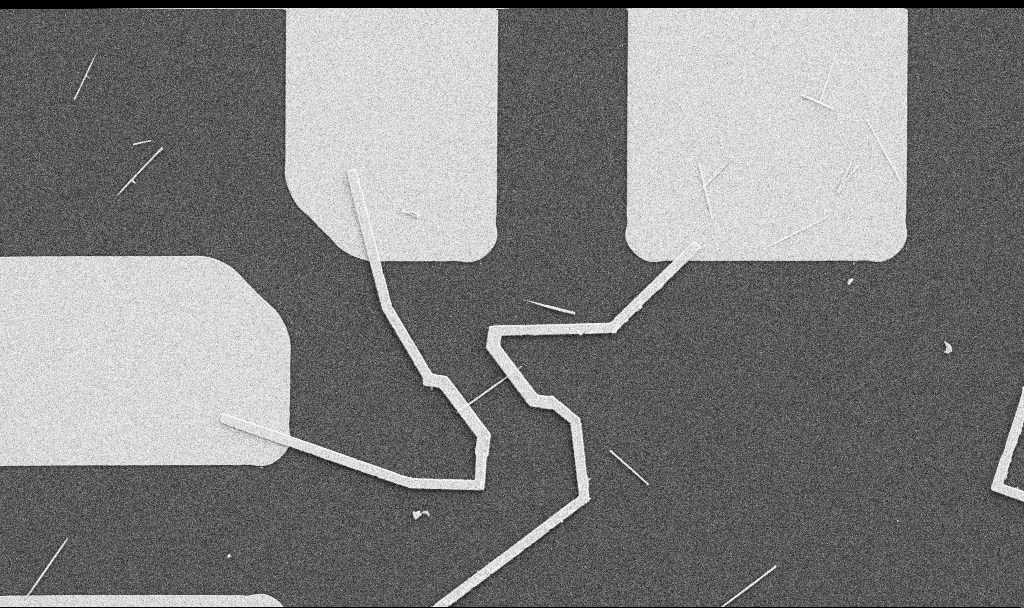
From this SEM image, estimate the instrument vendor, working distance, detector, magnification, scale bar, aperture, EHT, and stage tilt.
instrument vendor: Zeiss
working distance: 10.7 mm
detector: SE2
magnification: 5 K X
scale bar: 10000 nm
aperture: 30 µm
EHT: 5 kV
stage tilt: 0°